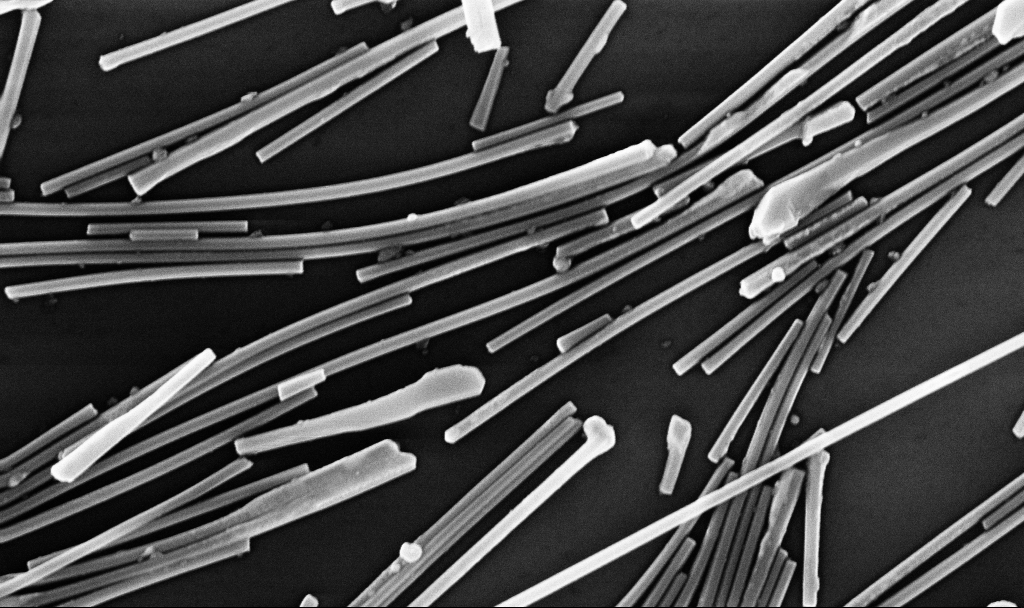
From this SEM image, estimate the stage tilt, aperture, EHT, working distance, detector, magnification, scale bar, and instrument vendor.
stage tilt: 0°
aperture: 30 µm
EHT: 10 kV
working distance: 6.7 mm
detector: InLens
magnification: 44.93 K X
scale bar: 1000 nm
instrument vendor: Zeiss